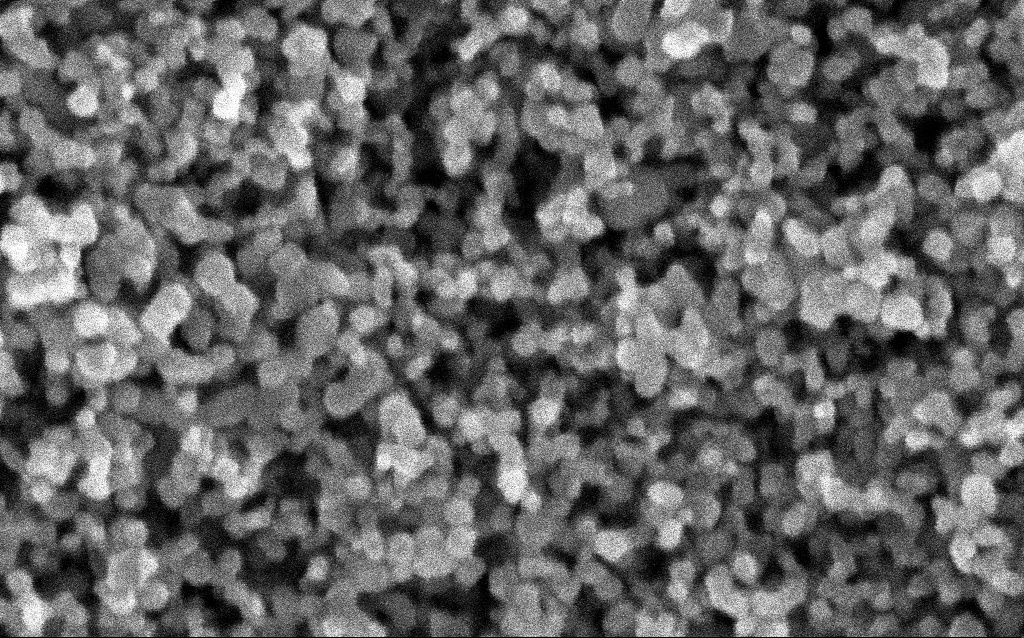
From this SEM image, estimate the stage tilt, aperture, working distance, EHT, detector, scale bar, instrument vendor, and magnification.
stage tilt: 0°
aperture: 30 µm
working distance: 4.9 mm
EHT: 5 kV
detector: InLens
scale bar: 100 nm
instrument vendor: Zeiss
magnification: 348.1 K X